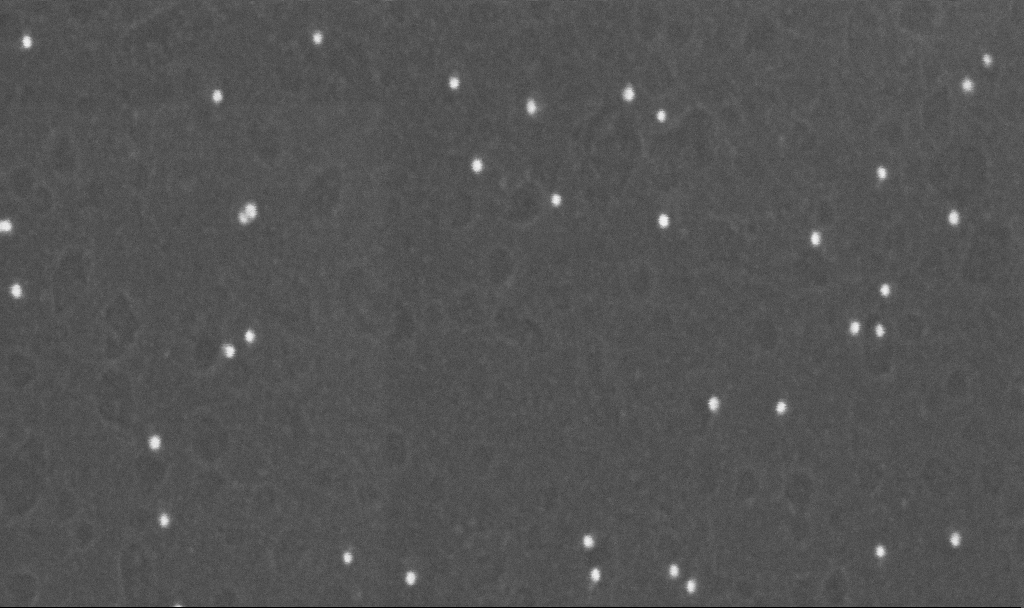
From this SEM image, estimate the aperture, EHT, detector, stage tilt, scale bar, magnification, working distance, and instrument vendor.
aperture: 30 µm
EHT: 10 kV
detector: InLens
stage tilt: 0°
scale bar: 100 nm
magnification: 350 K X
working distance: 3.1 mm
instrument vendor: Zeiss